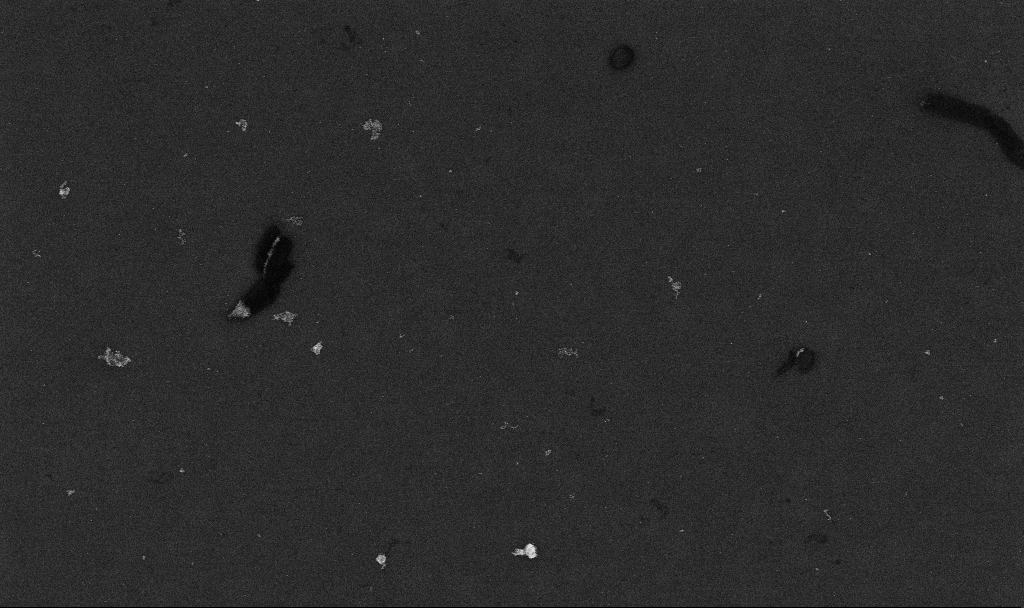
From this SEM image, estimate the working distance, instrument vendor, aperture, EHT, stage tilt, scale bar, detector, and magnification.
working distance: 3.3 mm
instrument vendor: Zeiss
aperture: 30 µm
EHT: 10 kV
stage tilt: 0°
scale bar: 10000 nm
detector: InLens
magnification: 6.98 K X